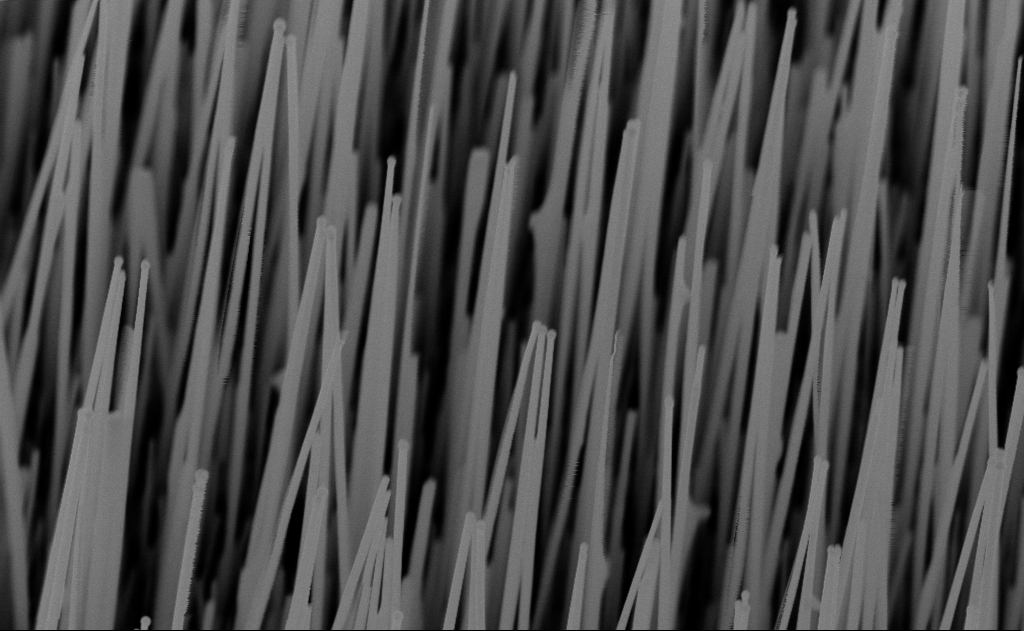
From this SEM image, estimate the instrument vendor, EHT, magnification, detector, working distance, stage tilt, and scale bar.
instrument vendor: Zeiss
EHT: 10 kV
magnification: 40 K X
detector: InLens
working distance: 8 mm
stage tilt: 30°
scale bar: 1000 nm